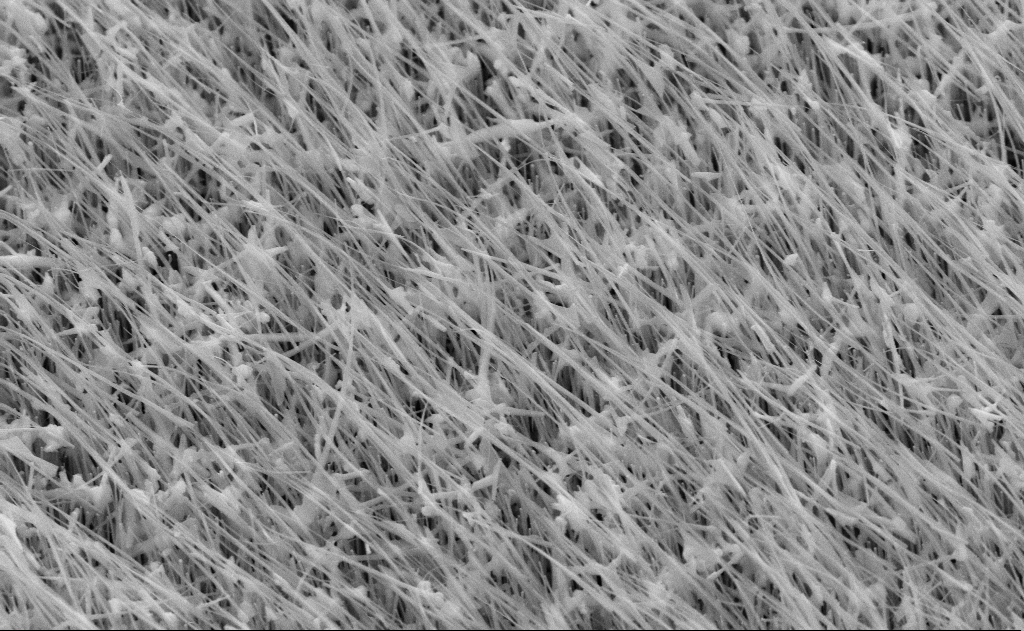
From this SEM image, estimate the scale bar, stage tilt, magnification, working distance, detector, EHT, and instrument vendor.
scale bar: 2000 nm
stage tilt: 45°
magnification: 30 K X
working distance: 12 mm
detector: SE2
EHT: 10 kV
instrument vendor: Zeiss